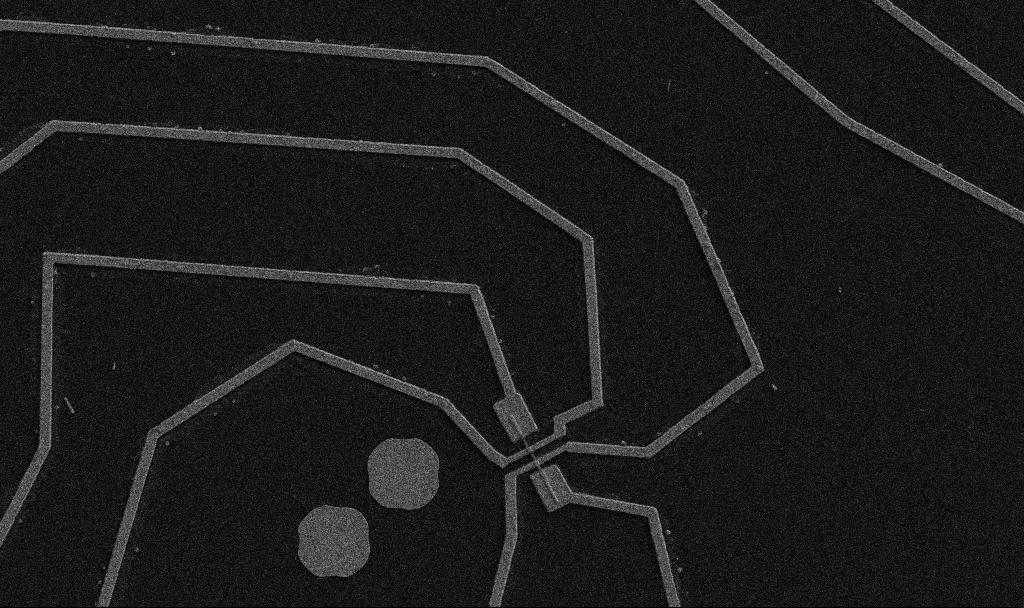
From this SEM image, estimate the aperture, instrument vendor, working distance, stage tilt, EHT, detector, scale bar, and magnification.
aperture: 30 µm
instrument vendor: Zeiss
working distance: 10.7 mm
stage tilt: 0°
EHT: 5 kV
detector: SE2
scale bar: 10000 nm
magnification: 5 K X